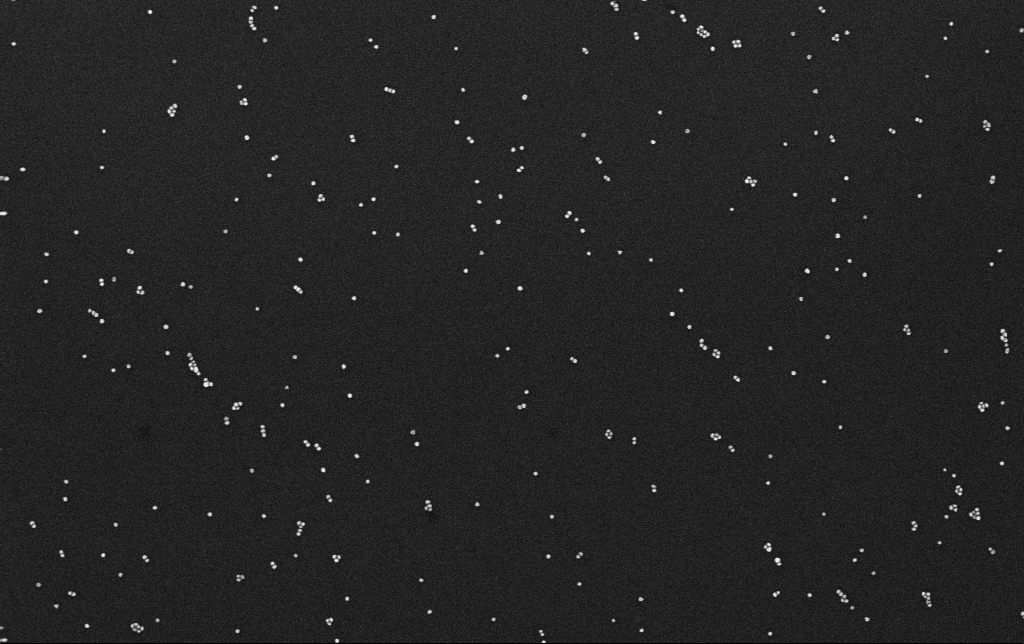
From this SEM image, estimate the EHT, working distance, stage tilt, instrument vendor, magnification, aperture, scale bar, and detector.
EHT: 10 kV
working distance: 3.1 mm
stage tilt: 0°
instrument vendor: Zeiss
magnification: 100 K X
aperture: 30 µm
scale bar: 200 nm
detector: InLens